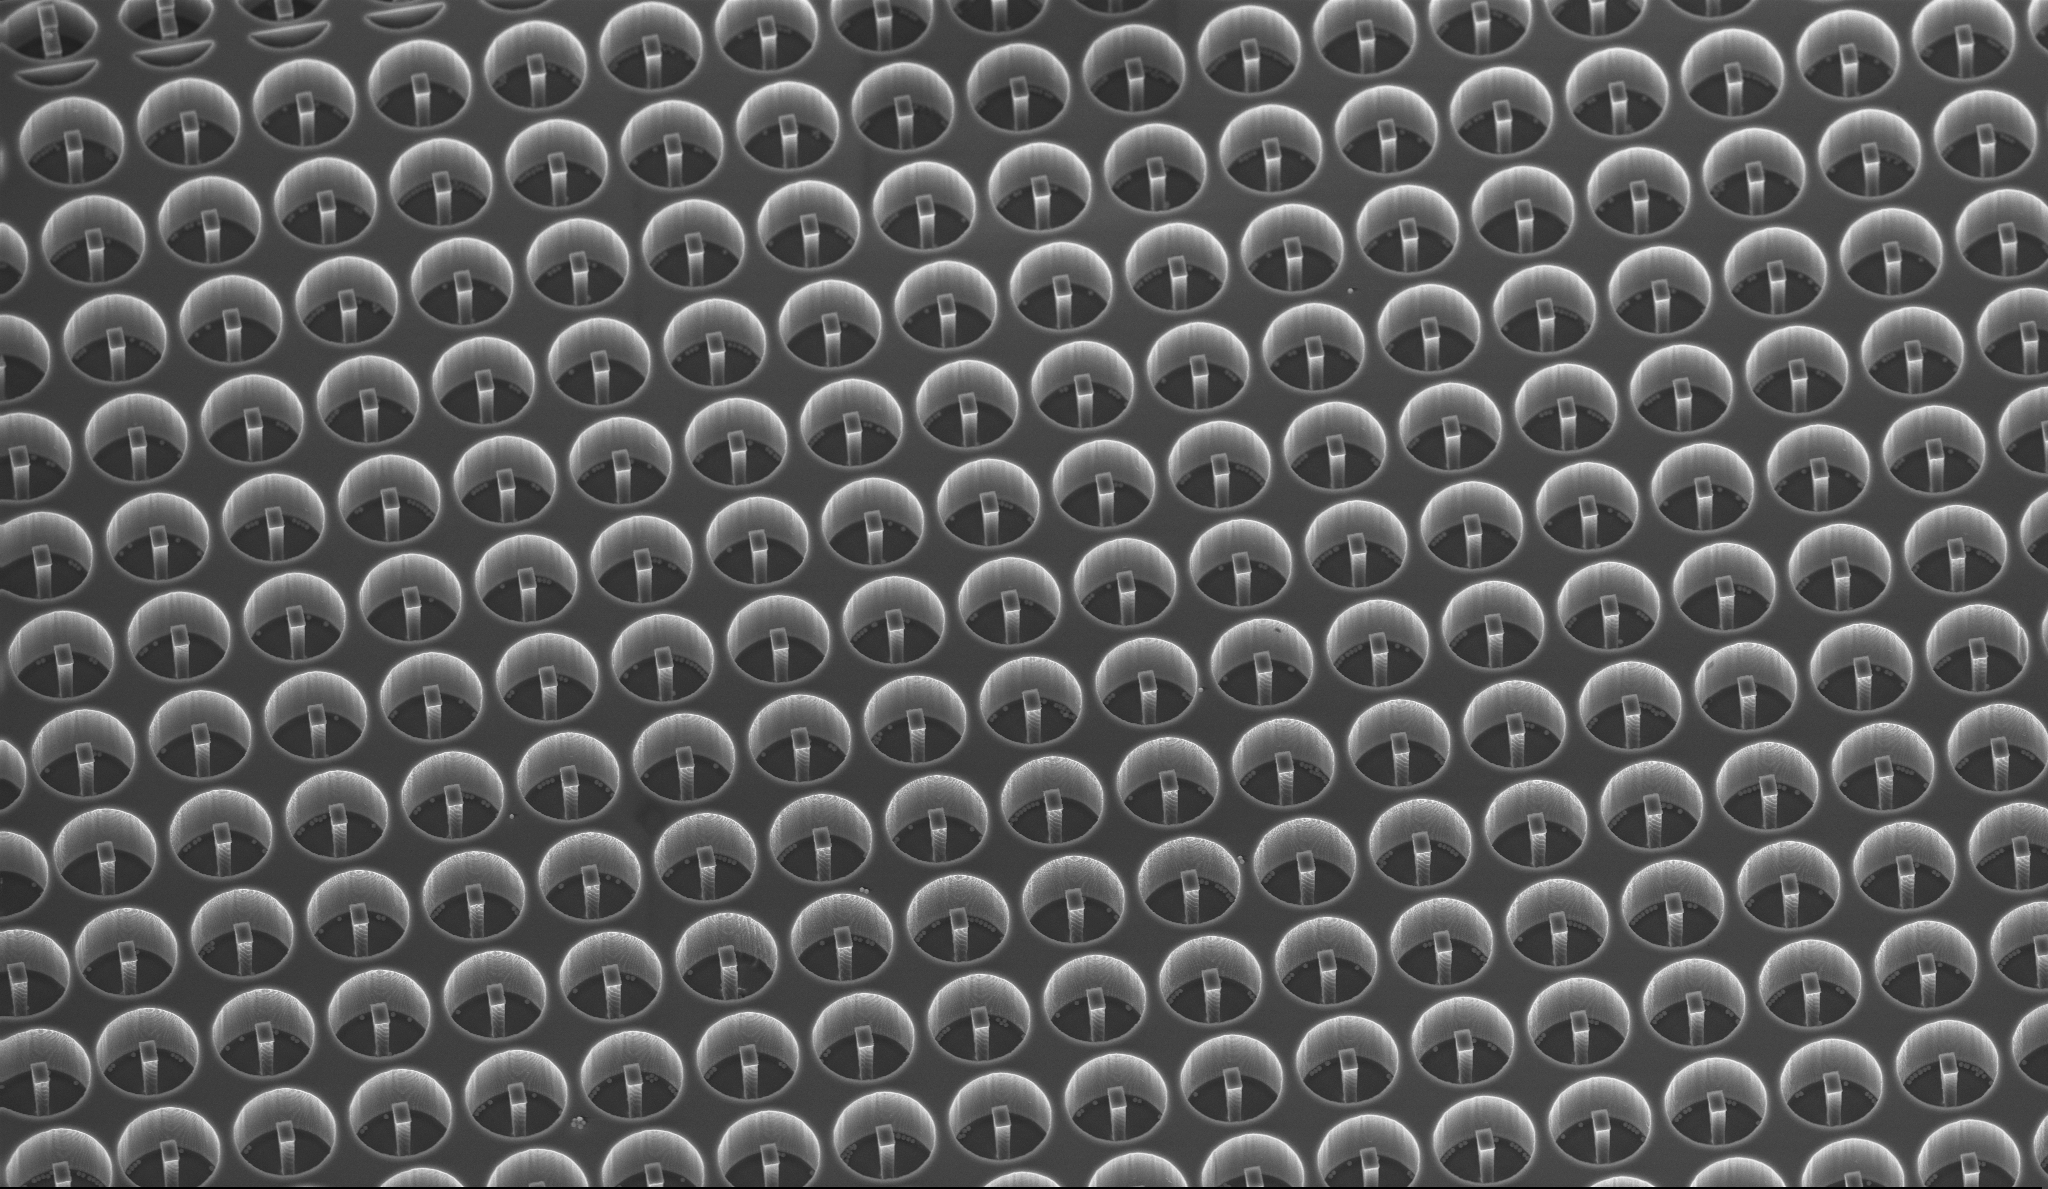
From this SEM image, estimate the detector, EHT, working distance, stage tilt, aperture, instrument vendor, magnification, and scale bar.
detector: InLens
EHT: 10 kV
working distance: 14.1 mm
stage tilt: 30°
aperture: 30 µm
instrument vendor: Zeiss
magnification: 1.52 K X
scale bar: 10000 nm